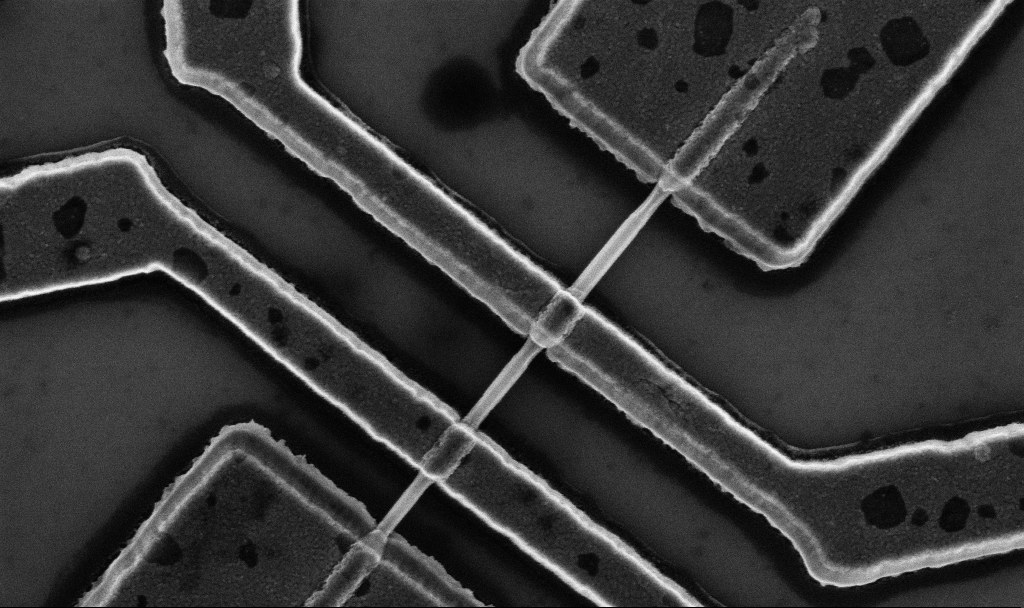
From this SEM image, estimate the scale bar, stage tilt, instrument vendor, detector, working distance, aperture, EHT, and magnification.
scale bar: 1000 nm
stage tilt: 0°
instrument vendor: Zeiss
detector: InLens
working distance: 10.7 mm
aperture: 30 µm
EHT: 5 kV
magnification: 60 K X